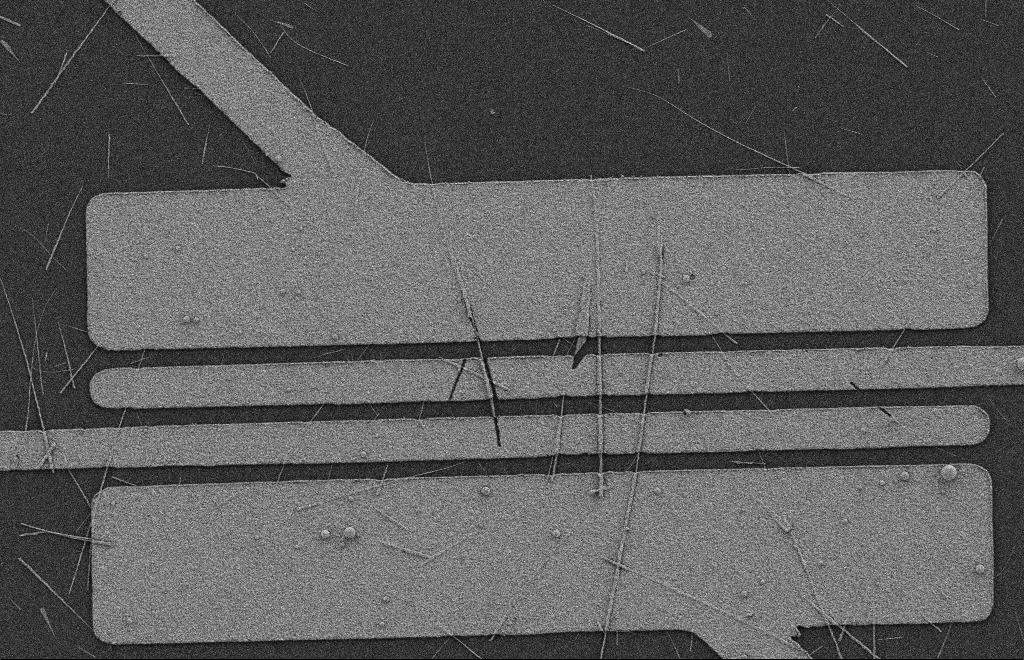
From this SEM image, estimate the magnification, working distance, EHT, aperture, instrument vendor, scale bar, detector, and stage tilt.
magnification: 5.4 K X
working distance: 9 mm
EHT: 2 kV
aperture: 20 µm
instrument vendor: Zeiss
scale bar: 2000 nm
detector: SE2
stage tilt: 0°